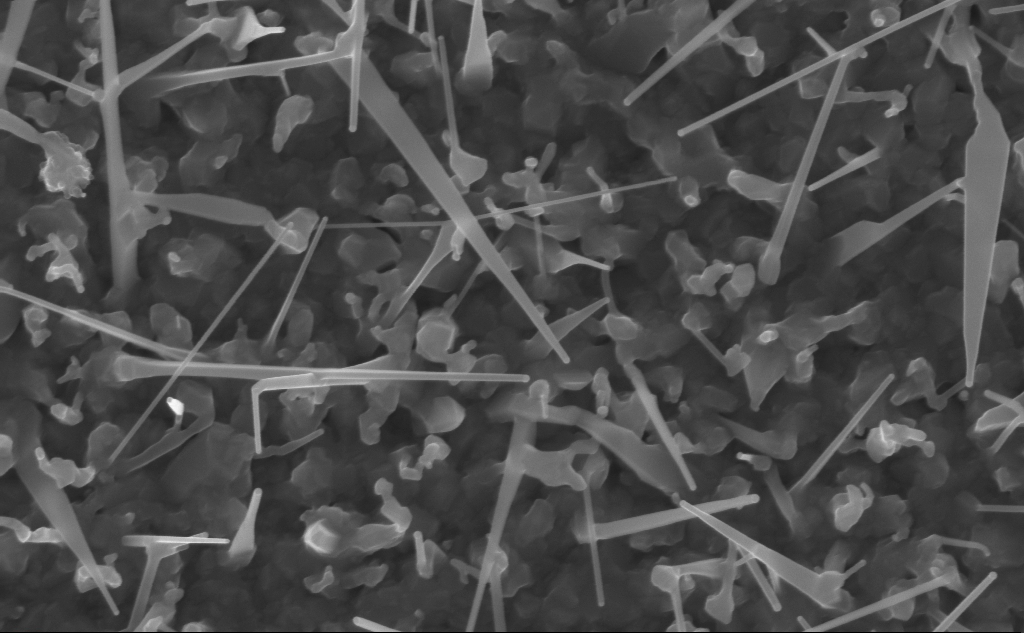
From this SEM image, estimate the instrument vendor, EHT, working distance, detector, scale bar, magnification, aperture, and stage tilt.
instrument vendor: Zeiss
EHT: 10 kV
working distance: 5 mm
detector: InLens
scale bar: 200 nm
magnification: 80 K X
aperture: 30 µm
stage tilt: -0°